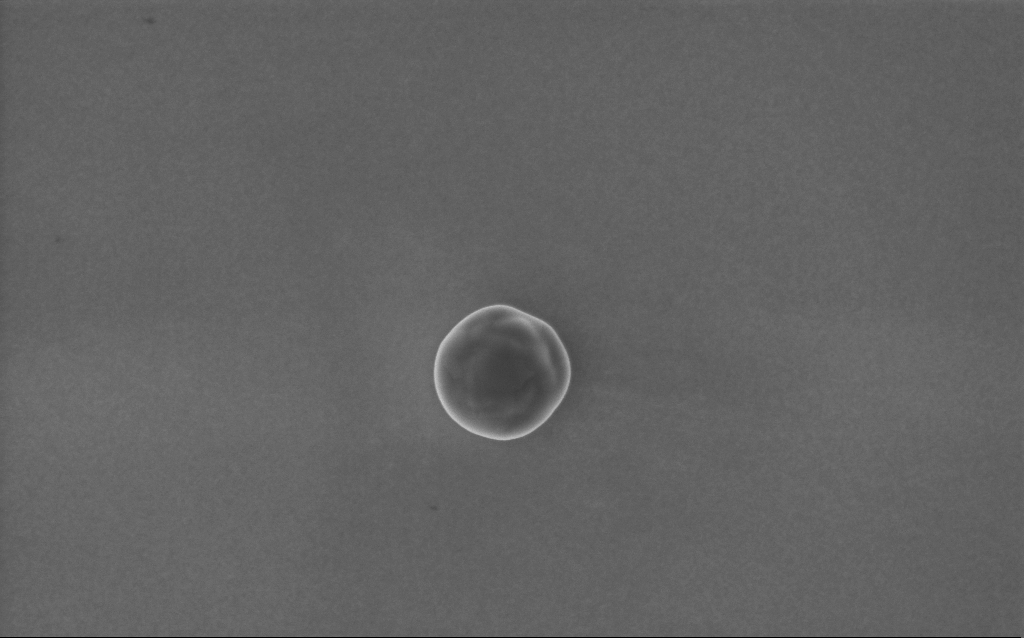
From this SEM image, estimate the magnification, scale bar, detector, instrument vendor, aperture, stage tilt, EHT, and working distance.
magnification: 46.88 K X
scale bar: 1000 nm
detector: InLens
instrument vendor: Zeiss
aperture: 30 µm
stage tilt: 0°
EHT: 5 kV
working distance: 4 mm